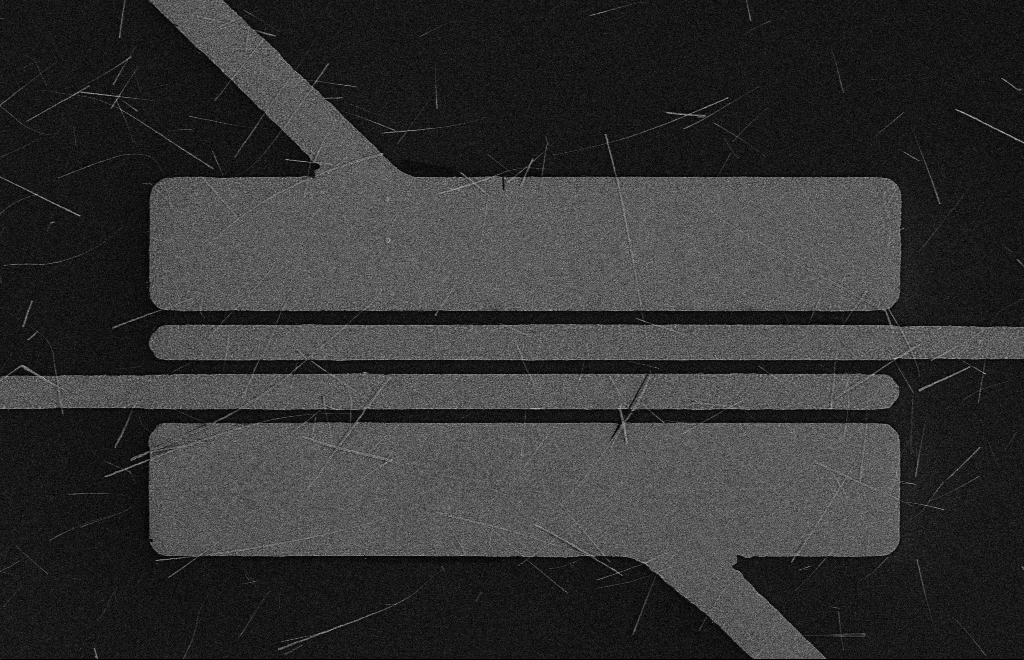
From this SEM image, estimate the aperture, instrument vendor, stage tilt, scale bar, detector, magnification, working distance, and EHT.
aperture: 10 µm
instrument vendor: Zeiss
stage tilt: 0°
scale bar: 10000 nm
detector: SE2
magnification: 4.53 K X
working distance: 16 mm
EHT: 5 kV